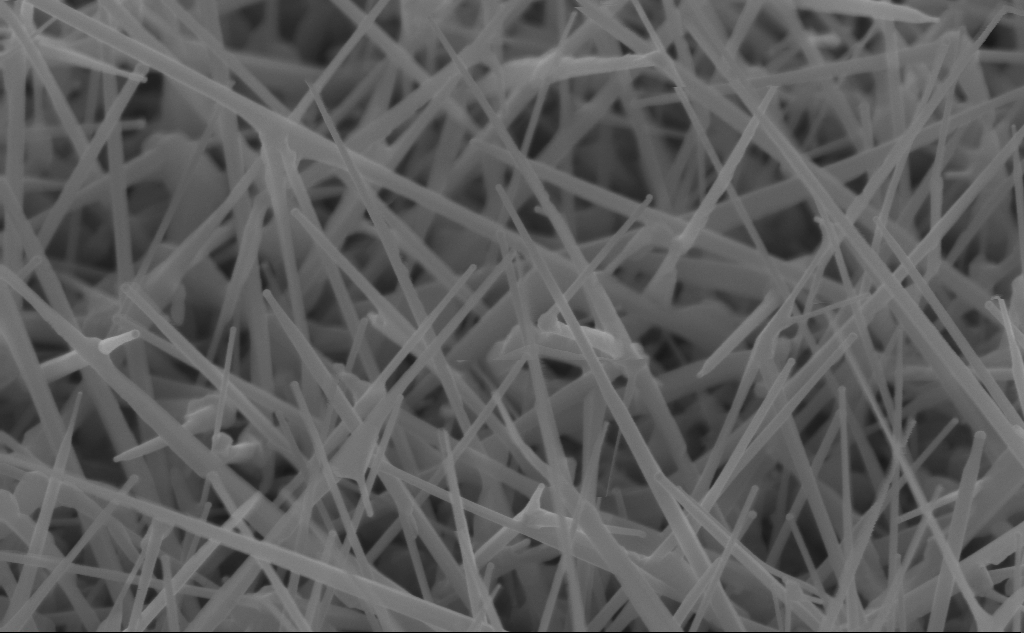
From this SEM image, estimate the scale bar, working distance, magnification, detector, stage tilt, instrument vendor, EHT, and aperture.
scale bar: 200 nm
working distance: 4 mm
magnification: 80 K X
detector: InLens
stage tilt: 45°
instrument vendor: Zeiss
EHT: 10 kV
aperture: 30 µm